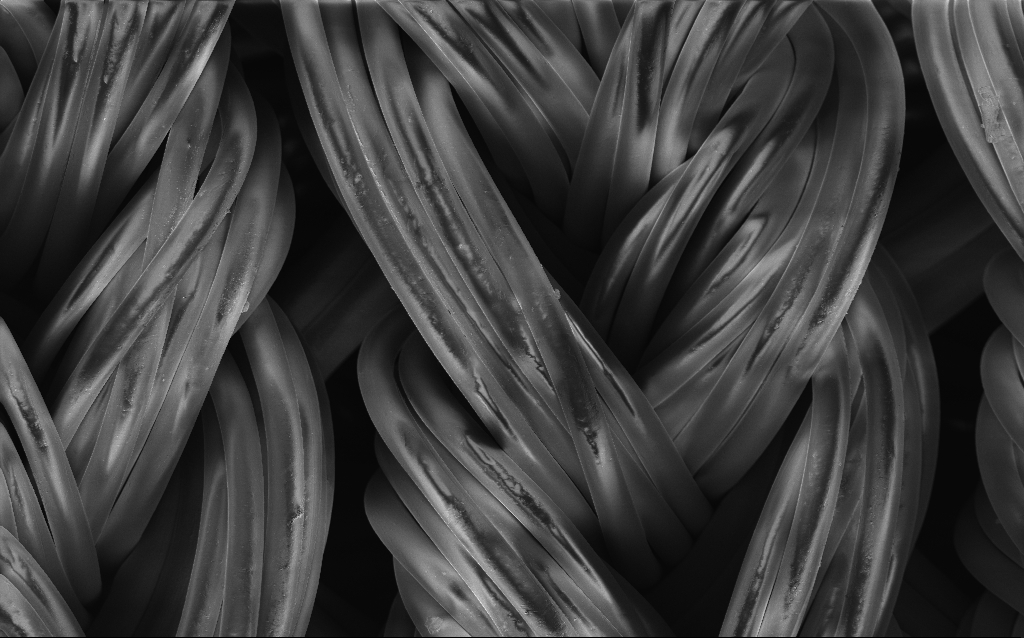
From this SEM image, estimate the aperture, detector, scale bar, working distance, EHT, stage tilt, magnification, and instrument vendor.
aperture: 30 µm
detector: InLens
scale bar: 100000 nm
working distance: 4 mm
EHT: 2 kV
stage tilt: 0°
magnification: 0.594 K X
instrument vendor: Zeiss